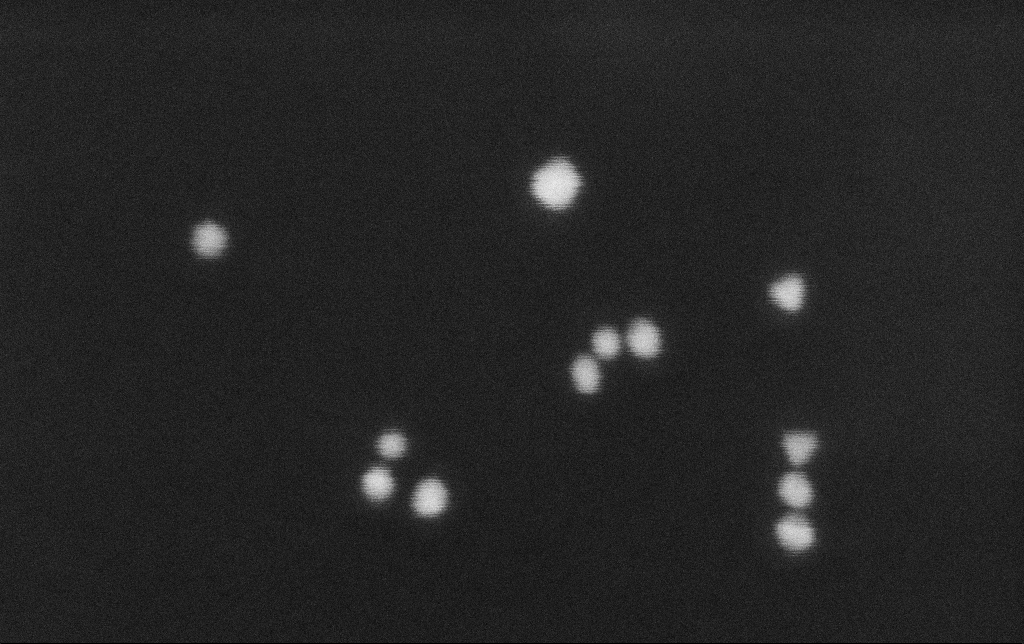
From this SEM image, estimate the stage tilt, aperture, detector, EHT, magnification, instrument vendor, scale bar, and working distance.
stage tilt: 0°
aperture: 30 µm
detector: SE2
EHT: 30 kV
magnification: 1000 K X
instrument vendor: Zeiss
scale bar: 20 nm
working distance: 10.8 mm